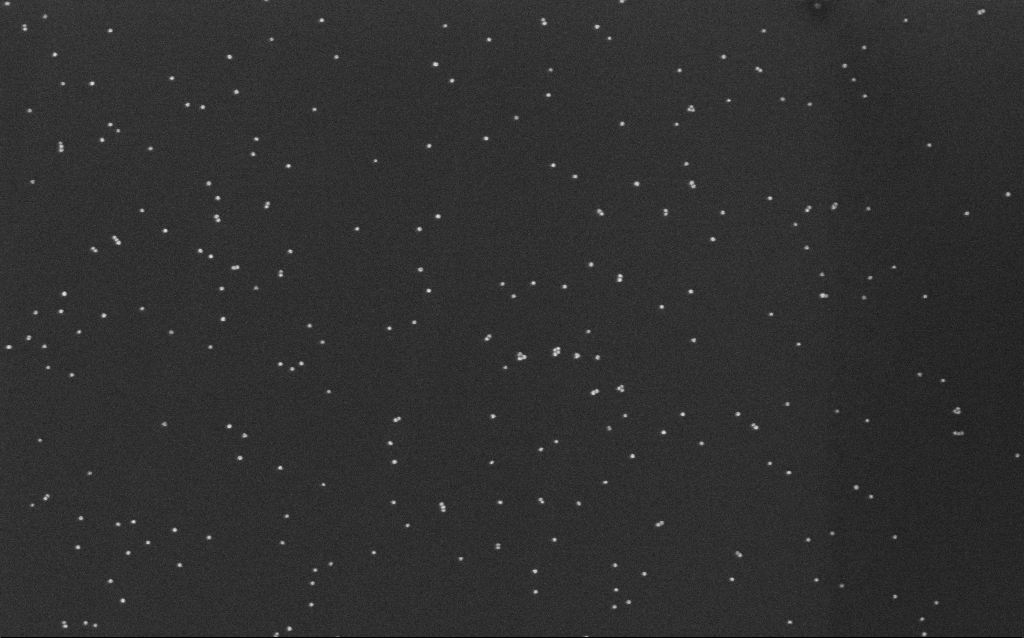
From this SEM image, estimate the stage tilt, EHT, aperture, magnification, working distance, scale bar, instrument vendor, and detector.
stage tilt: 0°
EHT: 10 kV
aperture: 30 µm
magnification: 100 K X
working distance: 6.6 mm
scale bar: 200 nm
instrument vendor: Zeiss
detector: InLens